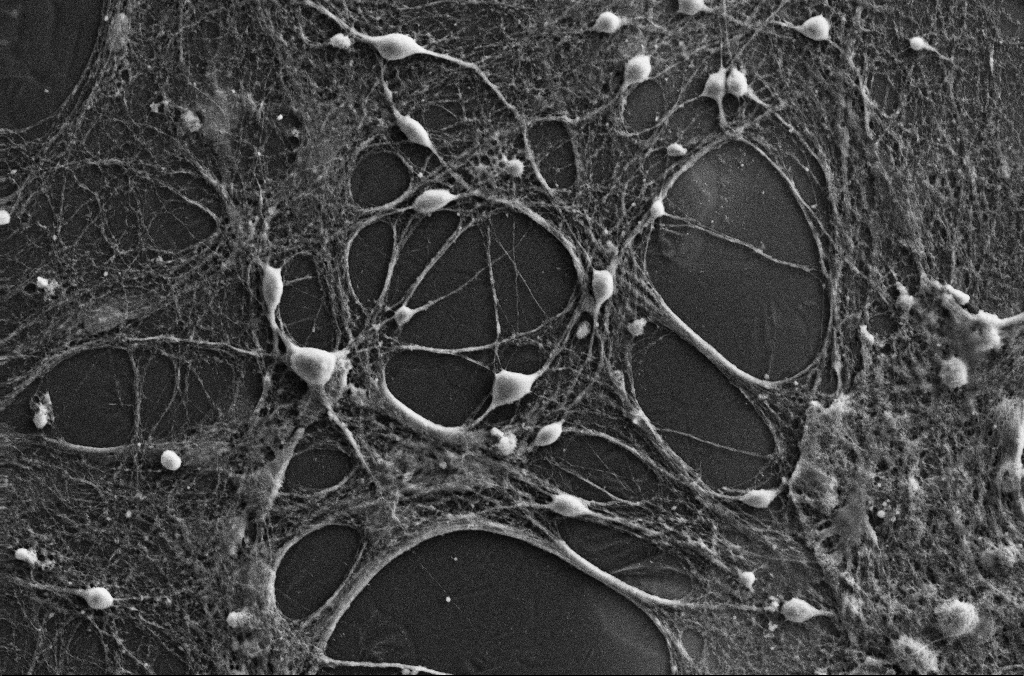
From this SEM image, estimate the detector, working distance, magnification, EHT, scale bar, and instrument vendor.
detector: SE2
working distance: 3.1 mm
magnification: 1 K X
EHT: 15 kV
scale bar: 20000 nm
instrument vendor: Zeiss